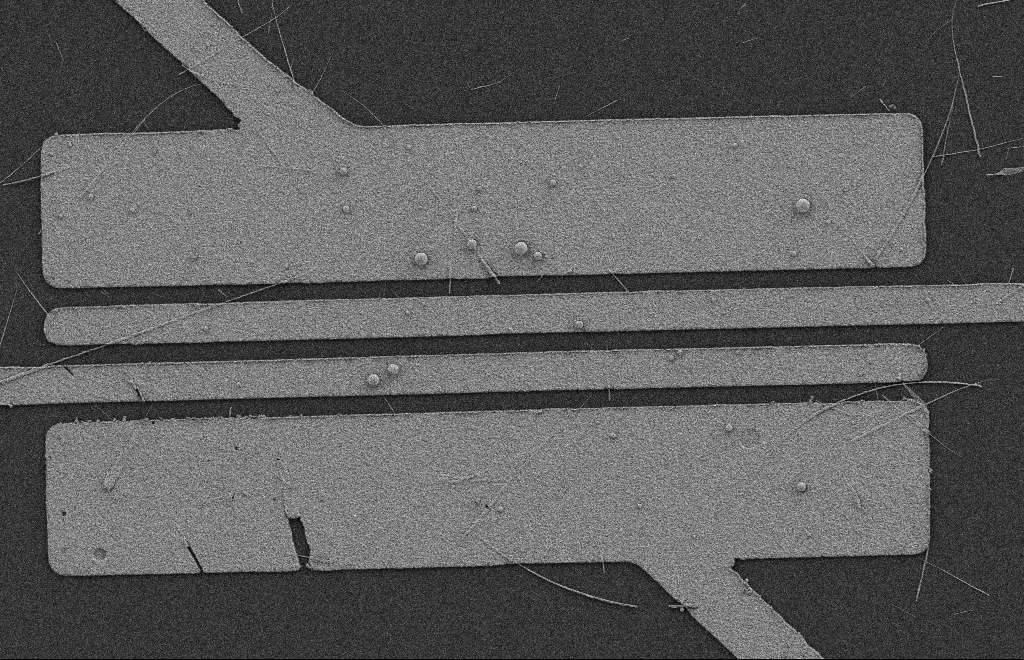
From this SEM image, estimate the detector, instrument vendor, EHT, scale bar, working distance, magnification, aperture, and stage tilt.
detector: SE2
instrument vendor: Zeiss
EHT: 2 kV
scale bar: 2000 nm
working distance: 9 mm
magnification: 5.29 K X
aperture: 20 µm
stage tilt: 0°